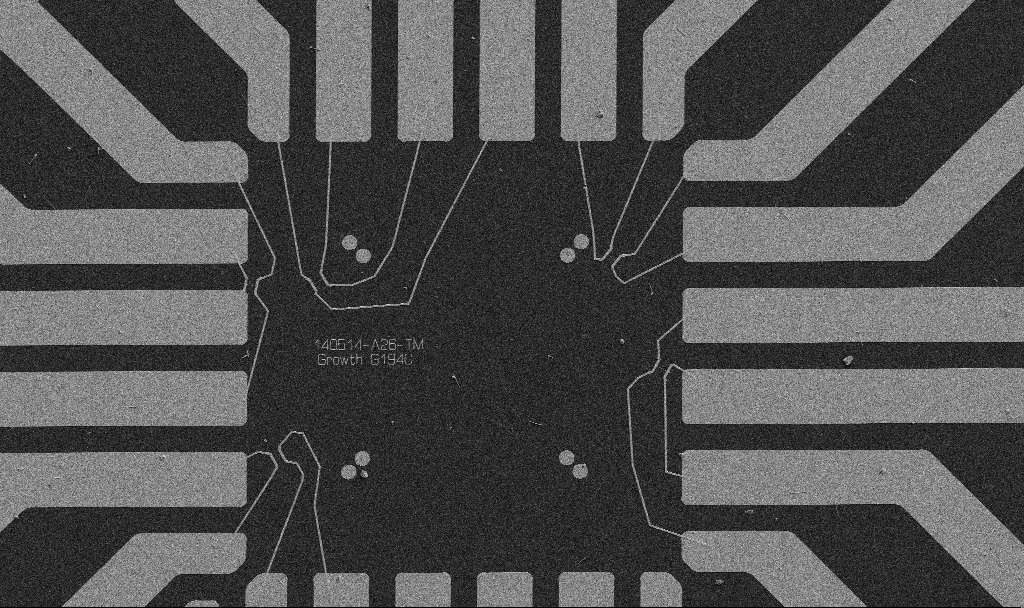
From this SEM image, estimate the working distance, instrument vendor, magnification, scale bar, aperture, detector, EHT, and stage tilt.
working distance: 10.7 mm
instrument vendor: Zeiss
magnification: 1 K X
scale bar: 20000 nm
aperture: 30 µm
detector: SE2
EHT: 5 kV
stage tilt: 0°